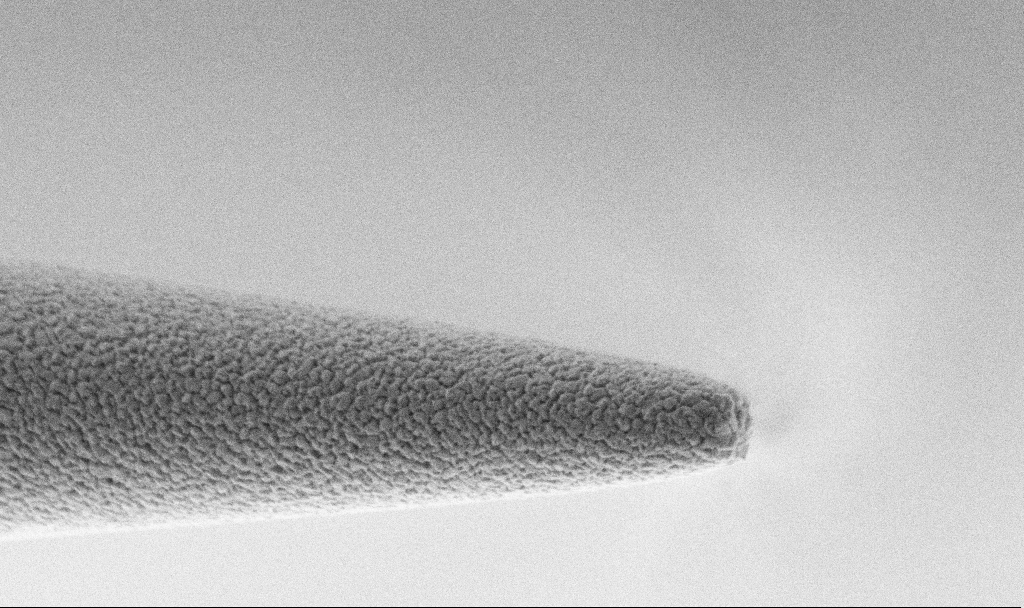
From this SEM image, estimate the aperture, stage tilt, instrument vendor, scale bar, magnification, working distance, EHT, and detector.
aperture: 30 µm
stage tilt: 70°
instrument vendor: Zeiss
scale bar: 1000 nm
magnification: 50 K X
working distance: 3.2 mm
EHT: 1.5 kV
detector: SE2